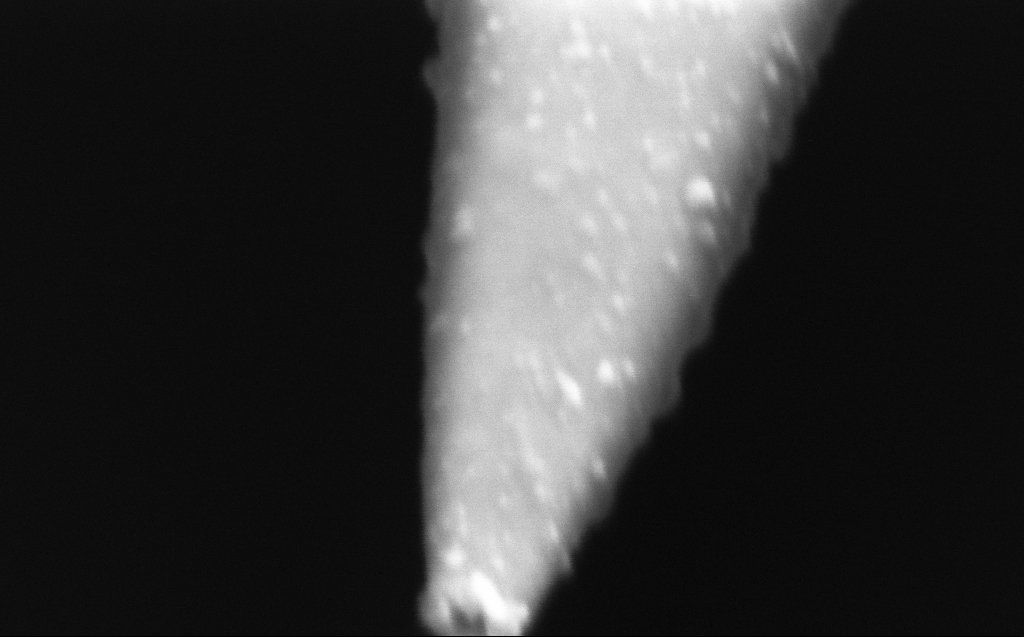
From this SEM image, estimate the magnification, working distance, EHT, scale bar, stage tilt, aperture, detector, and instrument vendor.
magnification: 331.66 K X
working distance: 5 mm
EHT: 2 kV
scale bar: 200 nm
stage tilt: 45°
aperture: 30 µm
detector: InLens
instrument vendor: Zeiss